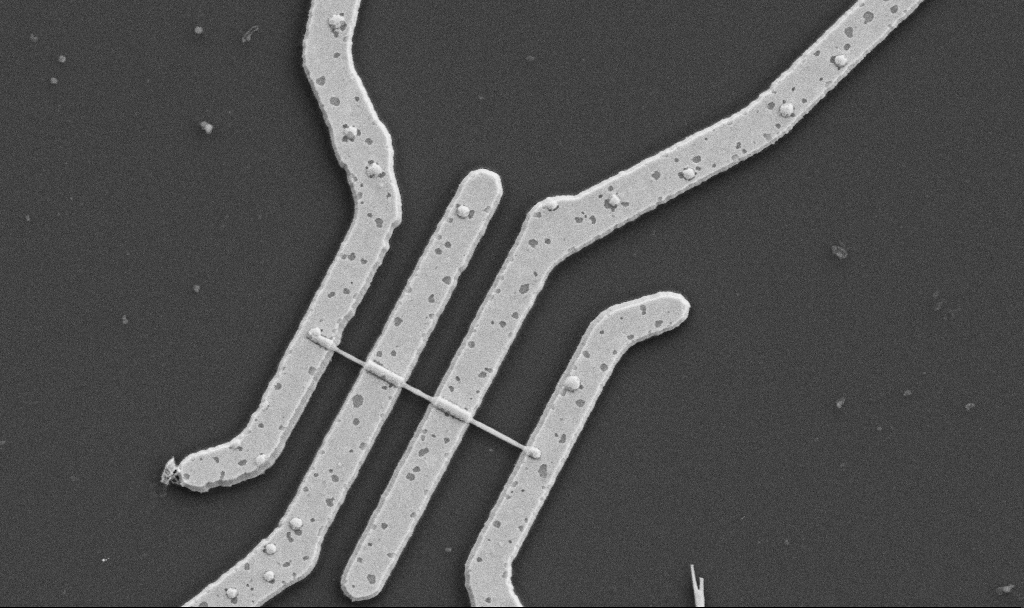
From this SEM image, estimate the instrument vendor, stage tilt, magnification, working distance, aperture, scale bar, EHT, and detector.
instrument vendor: Zeiss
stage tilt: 0°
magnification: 20 K X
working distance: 10.7 mm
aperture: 30 µm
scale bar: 1000 nm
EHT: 5 kV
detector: SE2